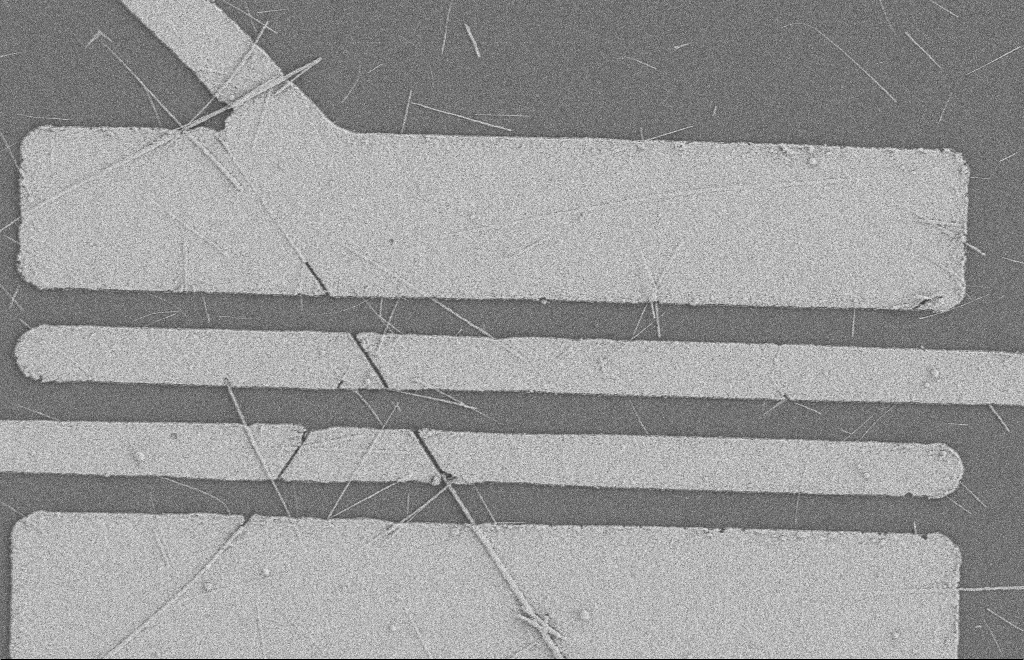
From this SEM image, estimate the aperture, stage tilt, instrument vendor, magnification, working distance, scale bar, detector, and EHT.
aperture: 20 µm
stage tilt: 0°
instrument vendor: Zeiss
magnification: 5.69 K X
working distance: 8 mm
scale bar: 2000 nm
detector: SE2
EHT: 2 kV